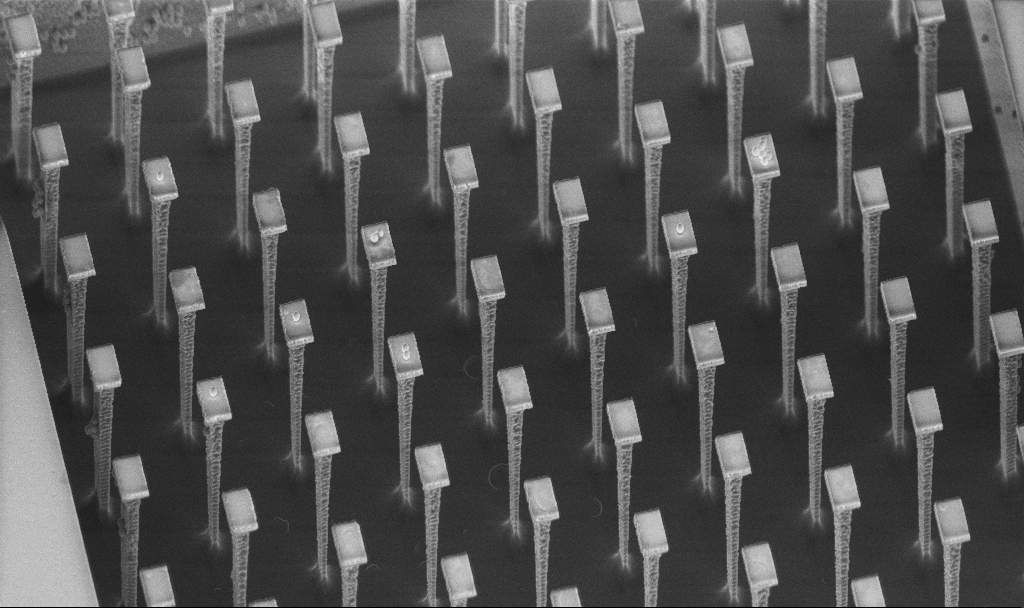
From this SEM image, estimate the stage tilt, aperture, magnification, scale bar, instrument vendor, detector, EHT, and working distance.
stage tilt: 45°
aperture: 30 µm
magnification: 3.48 K X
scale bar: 10000 nm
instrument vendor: Zeiss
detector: InLens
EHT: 5 kV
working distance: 4.3 mm